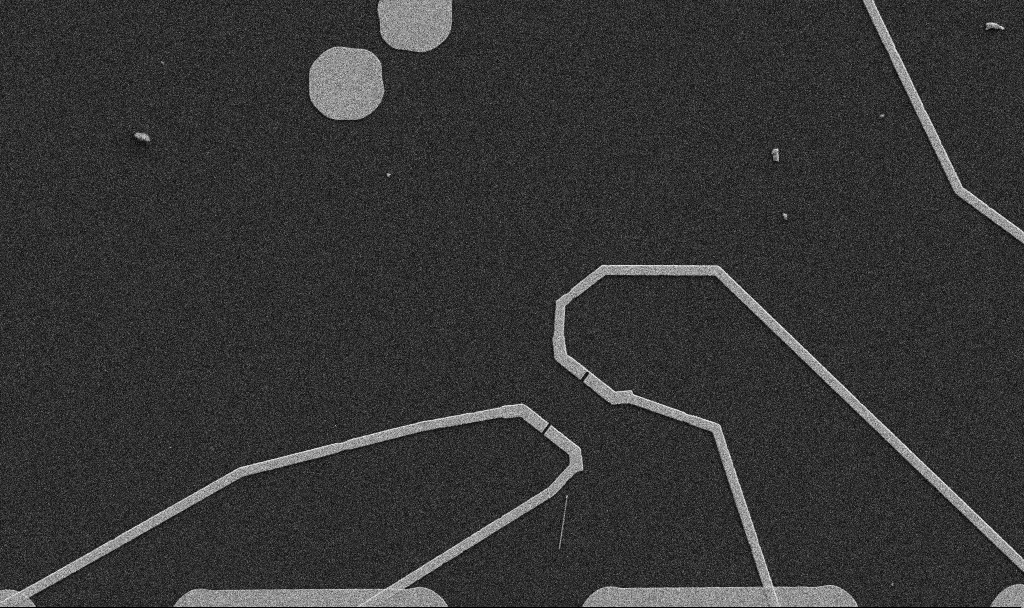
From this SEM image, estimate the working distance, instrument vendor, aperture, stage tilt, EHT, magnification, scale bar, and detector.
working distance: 10.7 mm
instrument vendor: Zeiss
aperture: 30 µm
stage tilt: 0°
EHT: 5 kV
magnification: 5 K X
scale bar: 10000 nm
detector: SE2